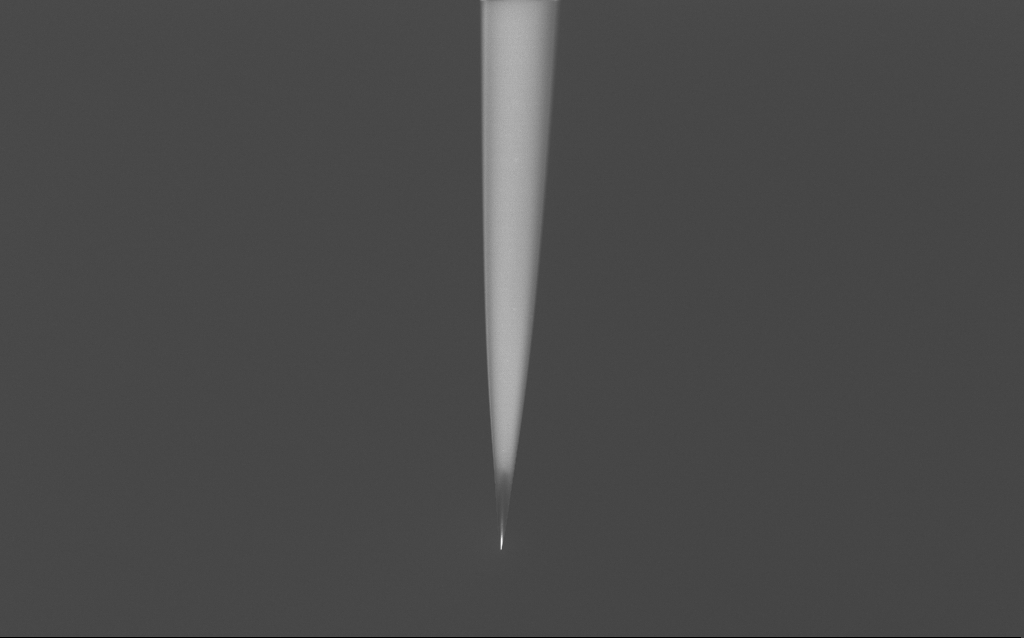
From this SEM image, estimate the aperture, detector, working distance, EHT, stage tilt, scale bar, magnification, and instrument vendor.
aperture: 30 µm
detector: InLens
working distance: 6 mm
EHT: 2 kV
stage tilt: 45°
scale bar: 20000 nm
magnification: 1 K X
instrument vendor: Zeiss